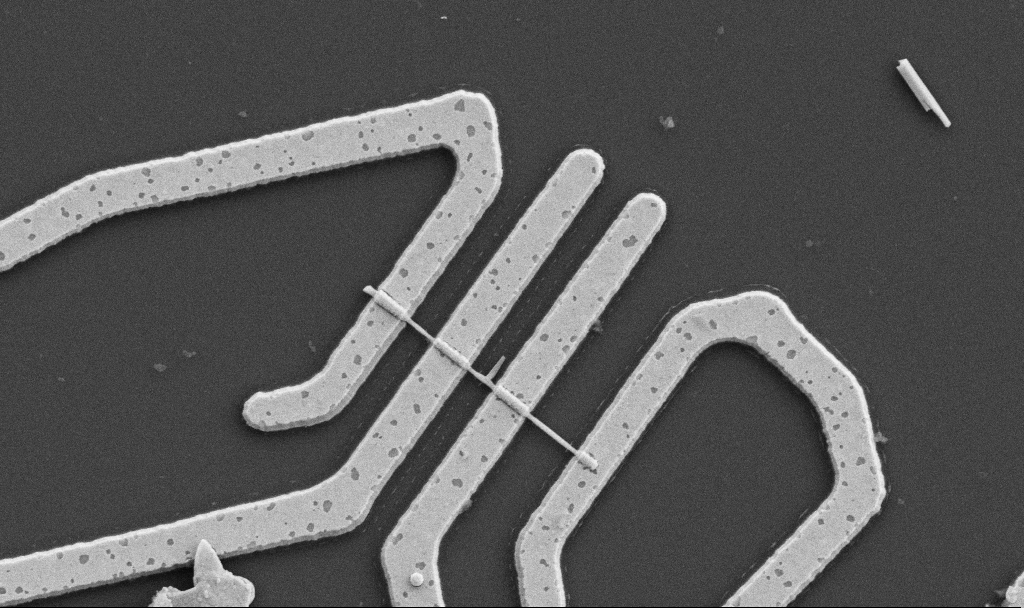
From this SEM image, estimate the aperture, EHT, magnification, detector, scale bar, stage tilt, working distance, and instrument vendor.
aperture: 30 µm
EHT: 5 kV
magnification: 20 K X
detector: SE2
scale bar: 1000 nm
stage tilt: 0°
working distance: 10.7 mm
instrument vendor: Zeiss